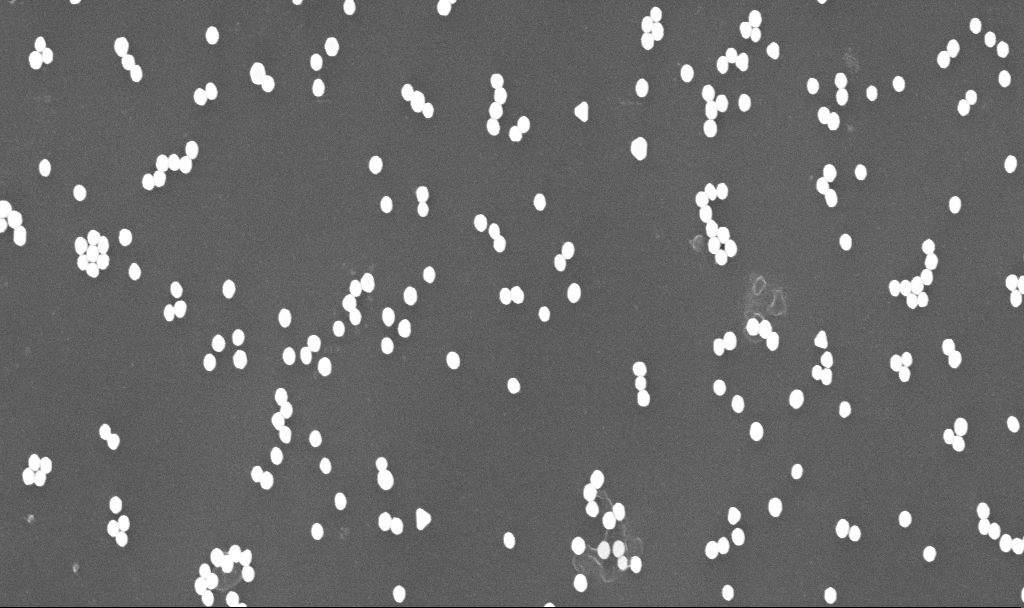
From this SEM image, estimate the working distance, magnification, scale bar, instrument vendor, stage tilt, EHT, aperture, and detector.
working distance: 3.4 mm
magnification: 70 K X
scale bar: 1000 nm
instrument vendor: Zeiss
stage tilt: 0°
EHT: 10 kV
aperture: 30 µm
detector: InLens